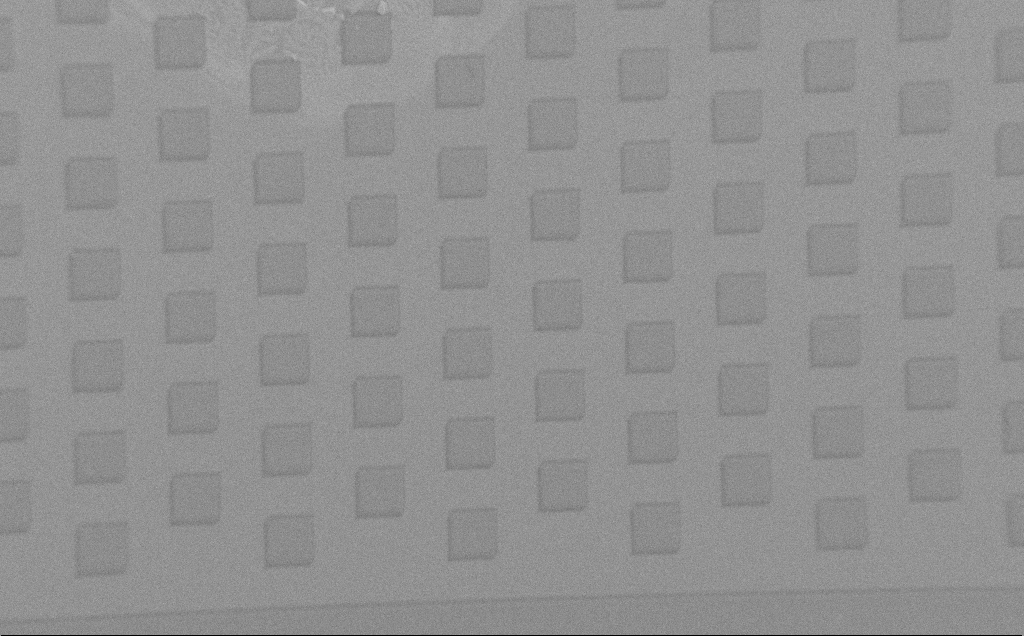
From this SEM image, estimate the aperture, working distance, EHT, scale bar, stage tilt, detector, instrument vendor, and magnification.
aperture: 30 µm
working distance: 7 mm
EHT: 1.5 kV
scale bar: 100000 nm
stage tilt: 0°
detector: SE2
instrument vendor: Zeiss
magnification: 0.332 K X